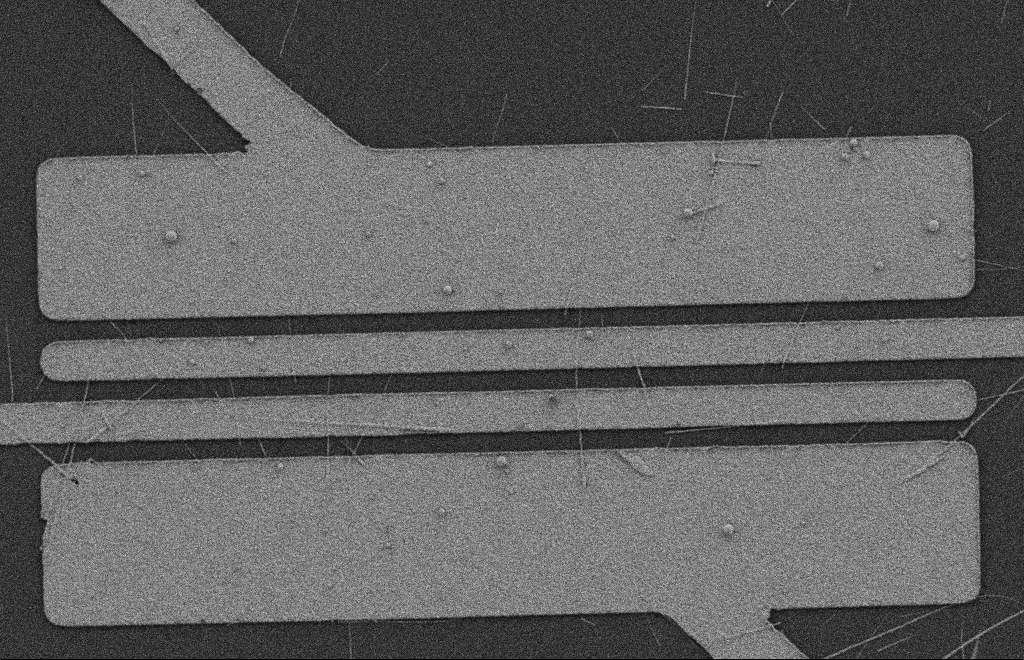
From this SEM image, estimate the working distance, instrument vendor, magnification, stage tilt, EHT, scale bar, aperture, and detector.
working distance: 9 mm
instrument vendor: Zeiss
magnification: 5.61 K X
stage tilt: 0°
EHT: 2 kV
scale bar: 2000 nm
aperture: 20 µm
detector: SE2